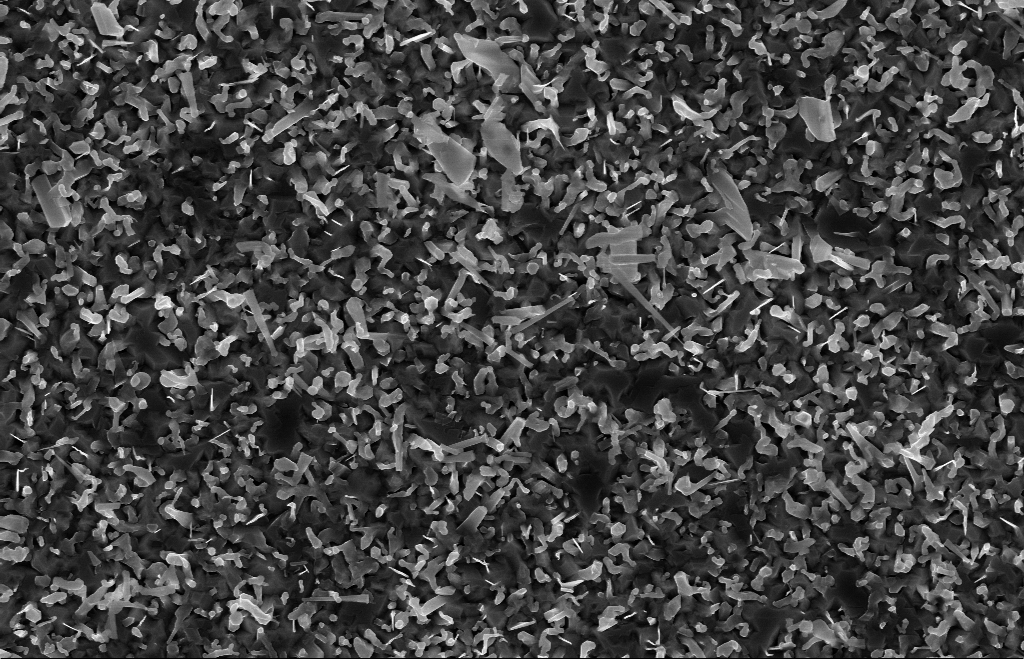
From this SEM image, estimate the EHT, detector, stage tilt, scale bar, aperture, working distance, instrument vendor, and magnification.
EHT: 10 kV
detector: InLens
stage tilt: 0°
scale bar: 1000 nm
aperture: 30 µm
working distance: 9 mm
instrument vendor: Zeiss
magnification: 20 K X